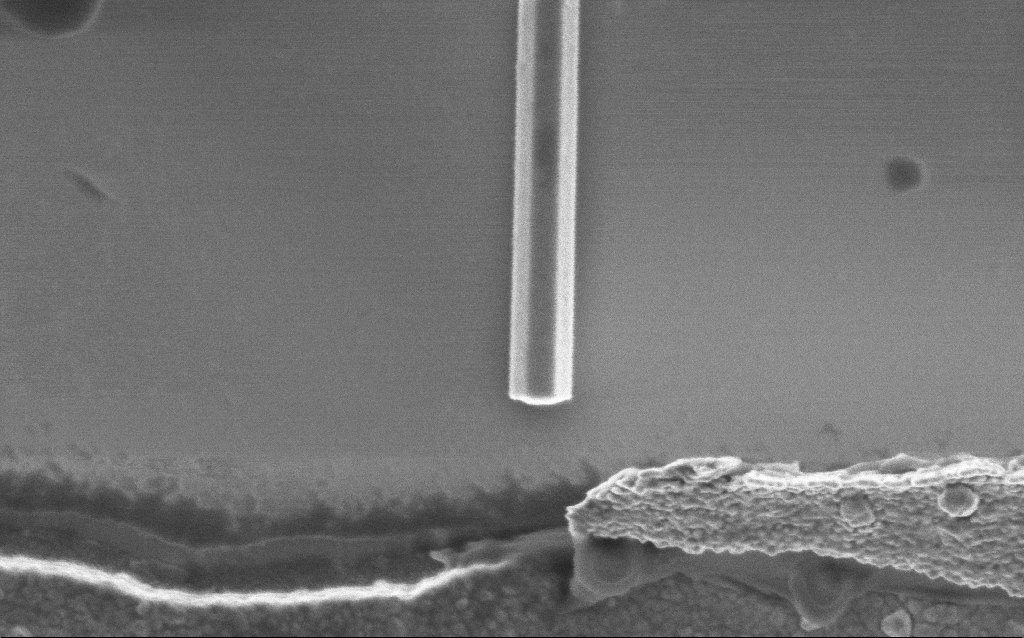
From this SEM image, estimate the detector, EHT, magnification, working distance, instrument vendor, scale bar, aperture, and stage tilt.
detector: InLens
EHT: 2 kV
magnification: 93.98 K X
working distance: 7.6 mm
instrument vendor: Zeiss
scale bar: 200 nm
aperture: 30 µm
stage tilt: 0°